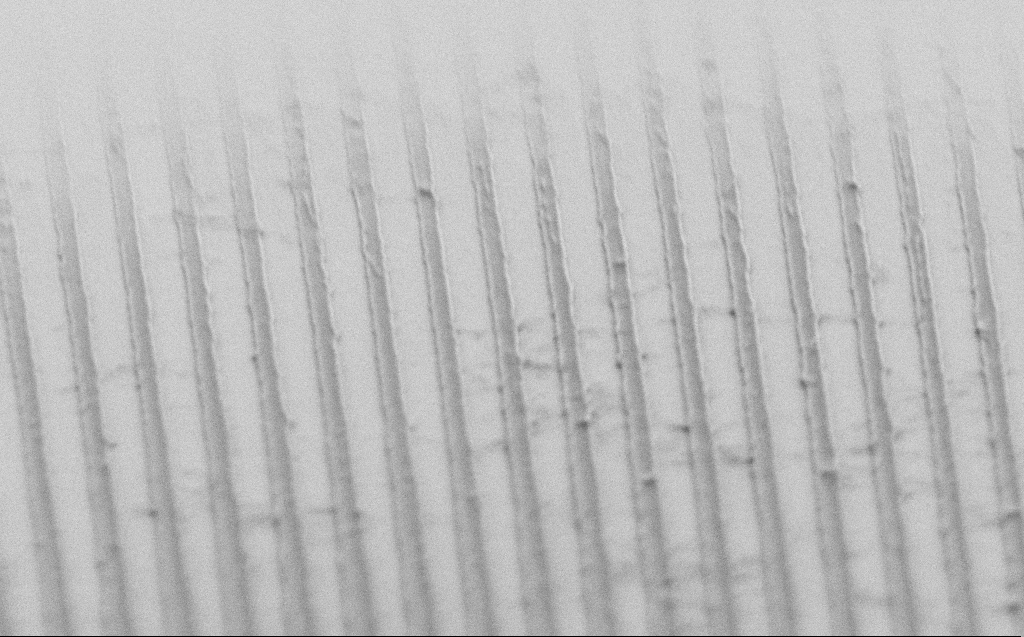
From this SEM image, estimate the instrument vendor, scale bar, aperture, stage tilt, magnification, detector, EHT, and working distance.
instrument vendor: Zeiss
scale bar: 1000 nm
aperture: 30 µm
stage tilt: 60.7°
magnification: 45.05 K X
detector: SE2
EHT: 1 kV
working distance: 3 mm